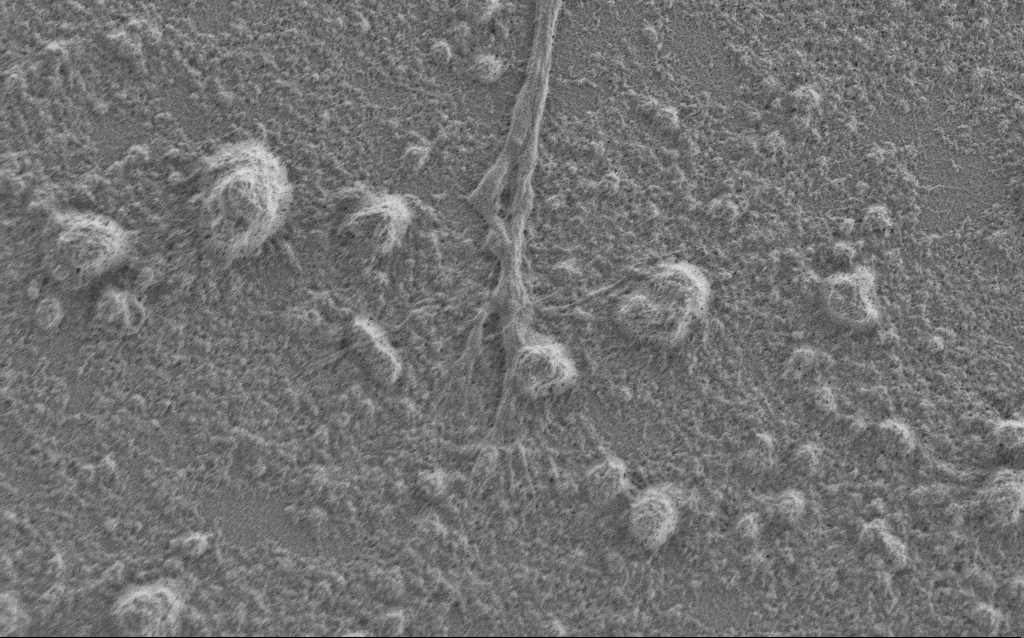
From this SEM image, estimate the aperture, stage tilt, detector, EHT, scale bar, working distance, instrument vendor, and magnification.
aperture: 30 µm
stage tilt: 0°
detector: SE2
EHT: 1 kV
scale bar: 2000 nm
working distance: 6 mm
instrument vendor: Zeiss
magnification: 7.5 K X